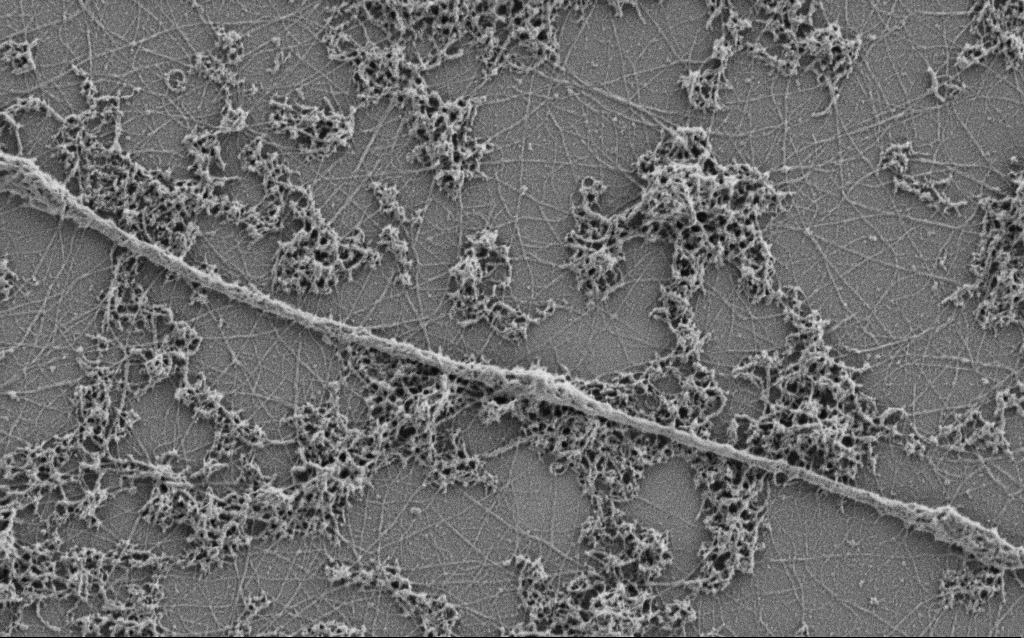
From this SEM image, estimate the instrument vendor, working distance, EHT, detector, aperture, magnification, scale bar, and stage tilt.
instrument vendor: Zeiss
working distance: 4 mm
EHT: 0.9 kV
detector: SE2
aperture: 30 µm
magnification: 20 K X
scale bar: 2000 nm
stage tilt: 0°